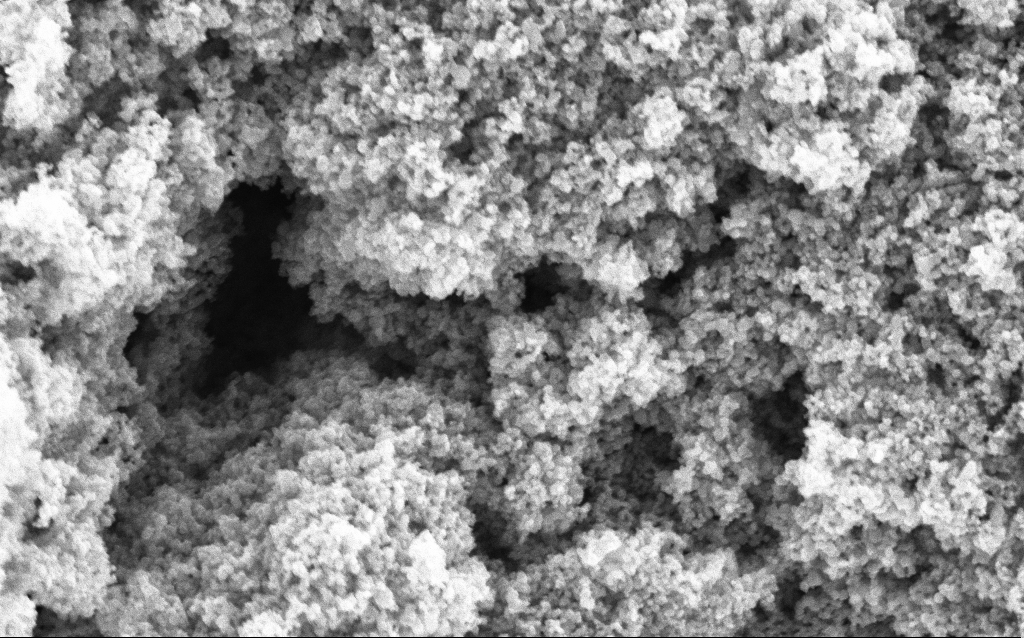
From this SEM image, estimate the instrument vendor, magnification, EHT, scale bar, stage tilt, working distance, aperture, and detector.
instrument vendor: Zeiss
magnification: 88.07 K X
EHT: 5 kV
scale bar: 200 nm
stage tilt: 0°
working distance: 4.6 mm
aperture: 30 µm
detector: InLens